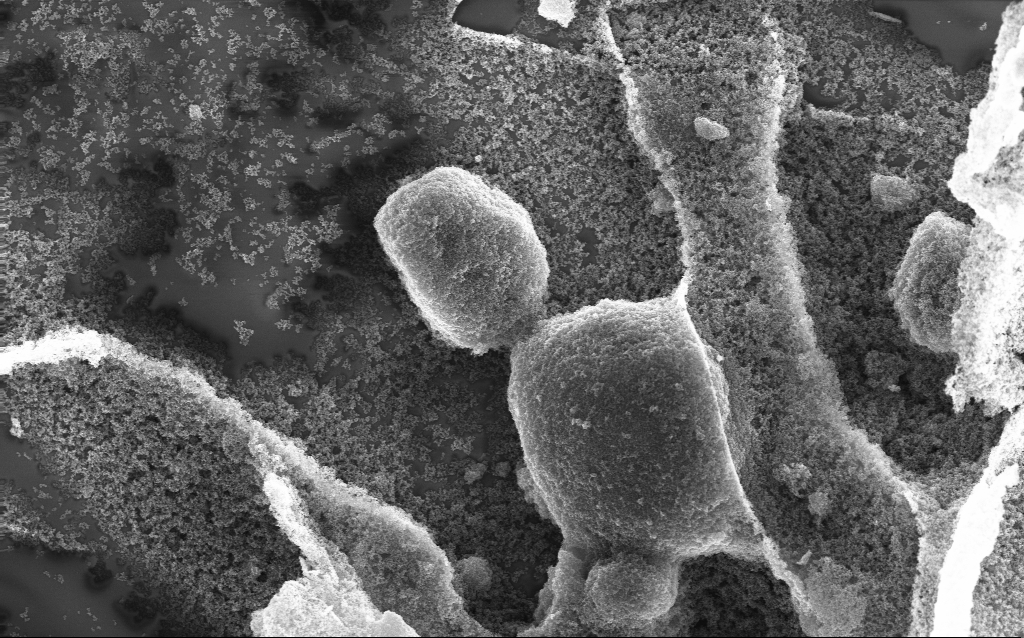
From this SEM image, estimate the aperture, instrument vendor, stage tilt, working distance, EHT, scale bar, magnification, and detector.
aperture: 30 µm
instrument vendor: Zeiss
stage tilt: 0°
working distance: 2.4 mm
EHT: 10 kV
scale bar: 1000 nm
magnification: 15.33 K X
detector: InLens